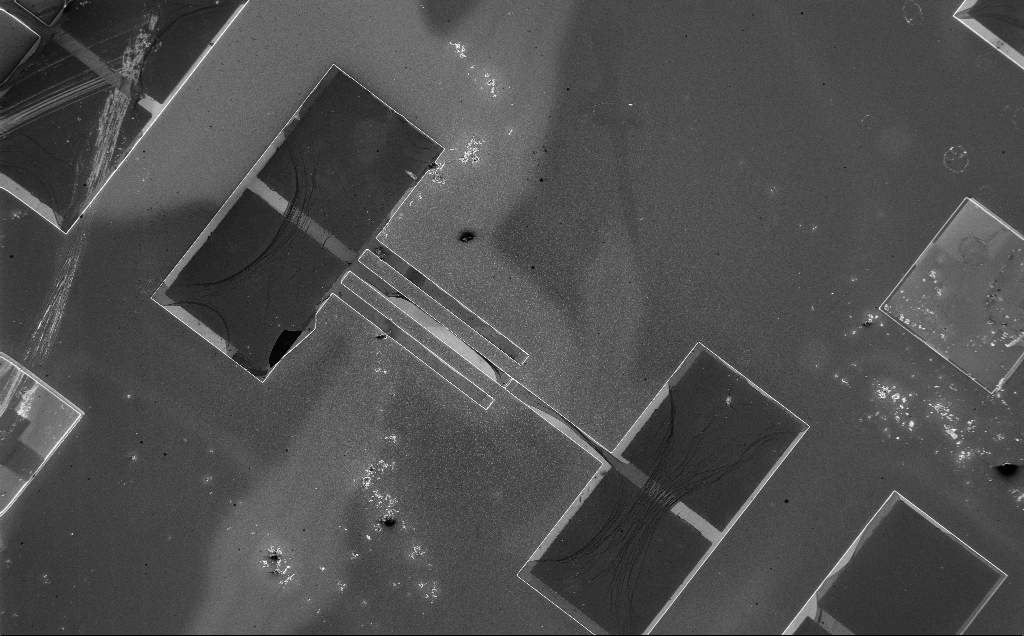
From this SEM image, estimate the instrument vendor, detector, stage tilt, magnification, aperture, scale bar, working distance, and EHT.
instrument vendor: Zeiss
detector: InLens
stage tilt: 0°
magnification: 0.262 K X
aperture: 30 µm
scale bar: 100000 nm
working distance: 10 mm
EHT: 5 kV